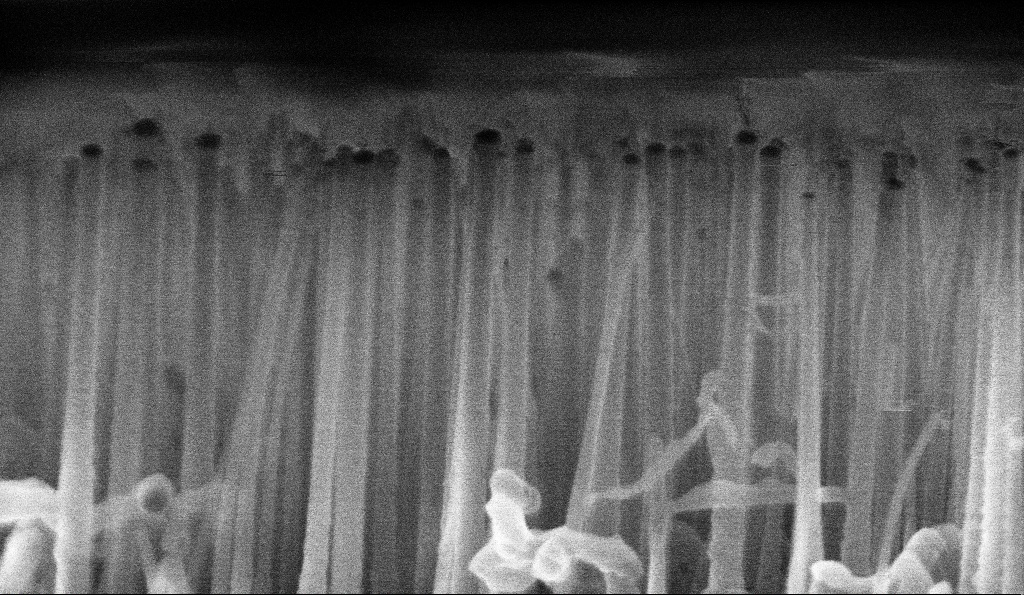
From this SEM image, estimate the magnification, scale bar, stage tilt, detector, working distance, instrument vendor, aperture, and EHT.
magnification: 214.52 K X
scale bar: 200 nm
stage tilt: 2.3°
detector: InLens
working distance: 3.3 mm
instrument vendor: Zeiss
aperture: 30 µm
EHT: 10 kV